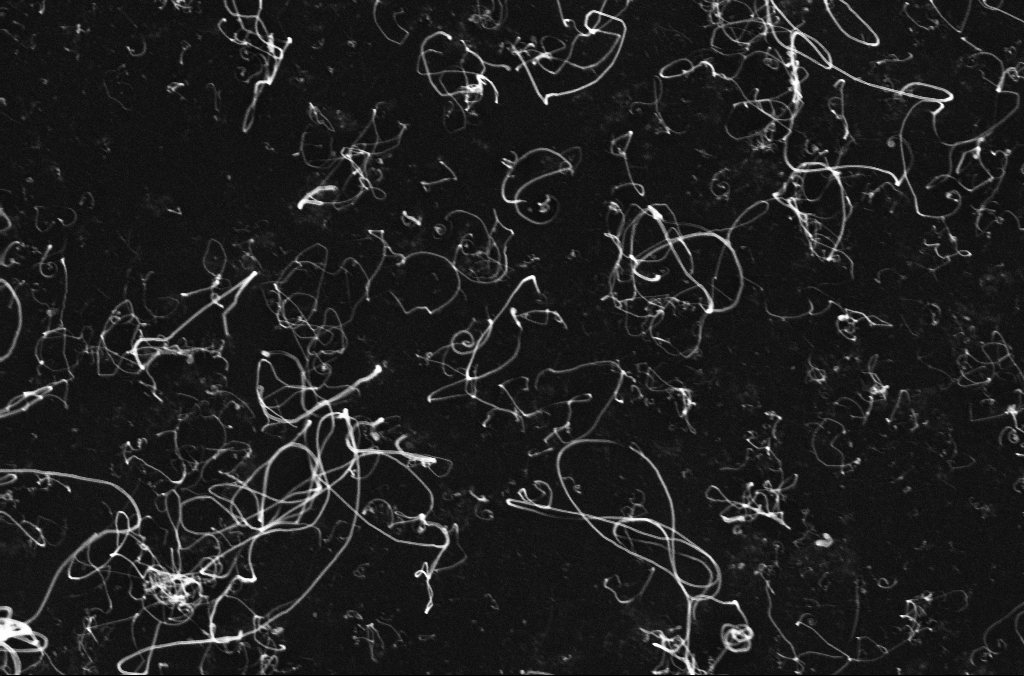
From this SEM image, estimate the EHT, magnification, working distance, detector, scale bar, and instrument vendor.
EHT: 10 kV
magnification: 50 K X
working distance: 3.3 mm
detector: InLens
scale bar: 1000 nm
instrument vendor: Zeiss